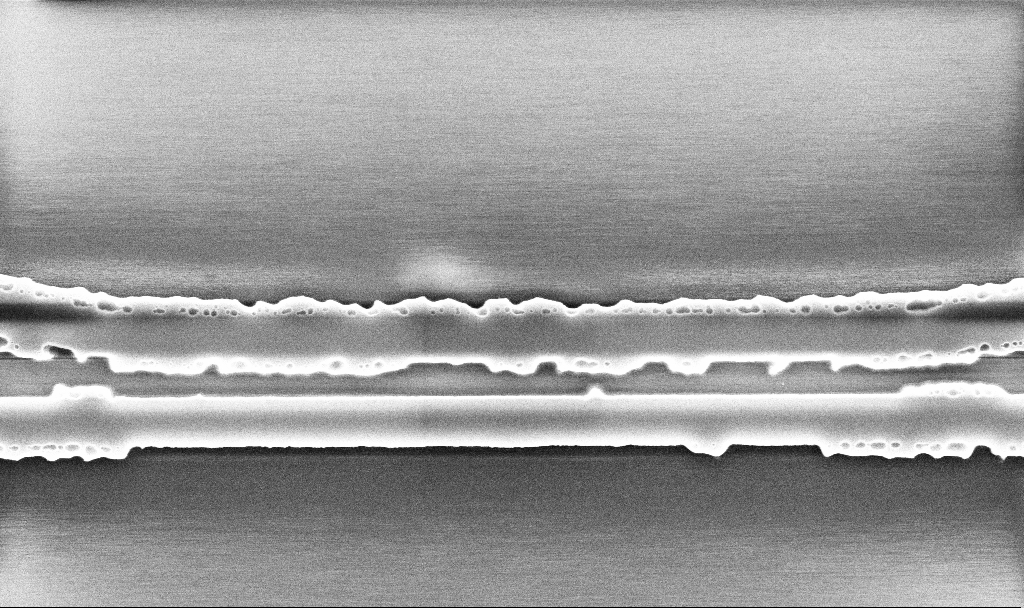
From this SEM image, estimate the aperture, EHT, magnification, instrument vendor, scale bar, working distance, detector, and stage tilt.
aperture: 30 µm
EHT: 5 kV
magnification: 37.38 K X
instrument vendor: Zeiss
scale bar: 1000 nm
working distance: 10.1 mm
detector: InLens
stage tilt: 0°